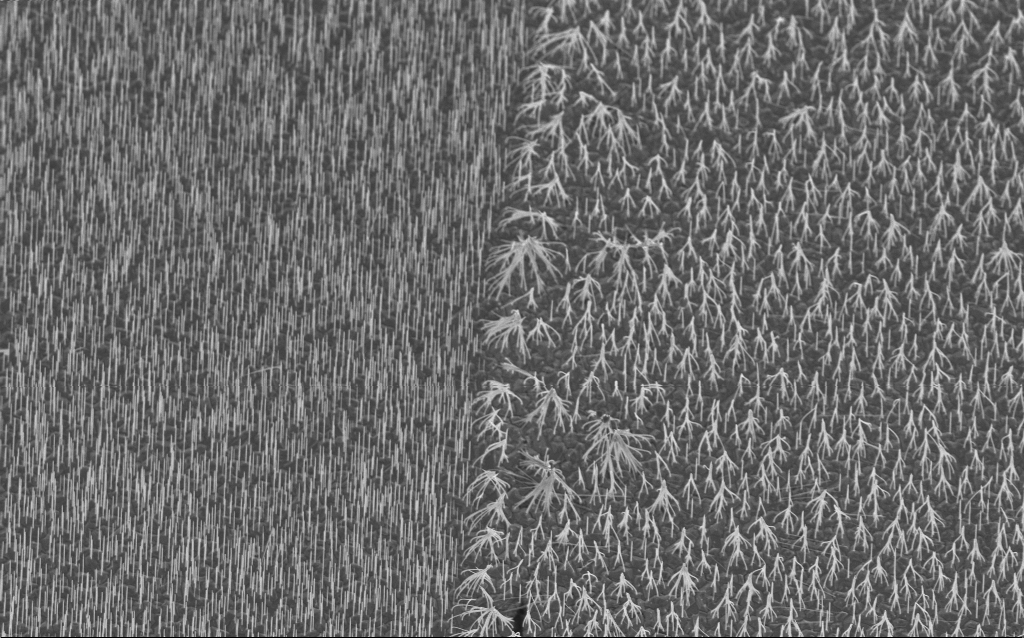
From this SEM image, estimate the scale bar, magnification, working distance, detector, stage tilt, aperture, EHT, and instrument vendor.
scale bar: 10000 nm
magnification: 5 K X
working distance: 6.5 mm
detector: InLens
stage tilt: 20°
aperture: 30 µm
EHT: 5 kV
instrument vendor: Zeiss